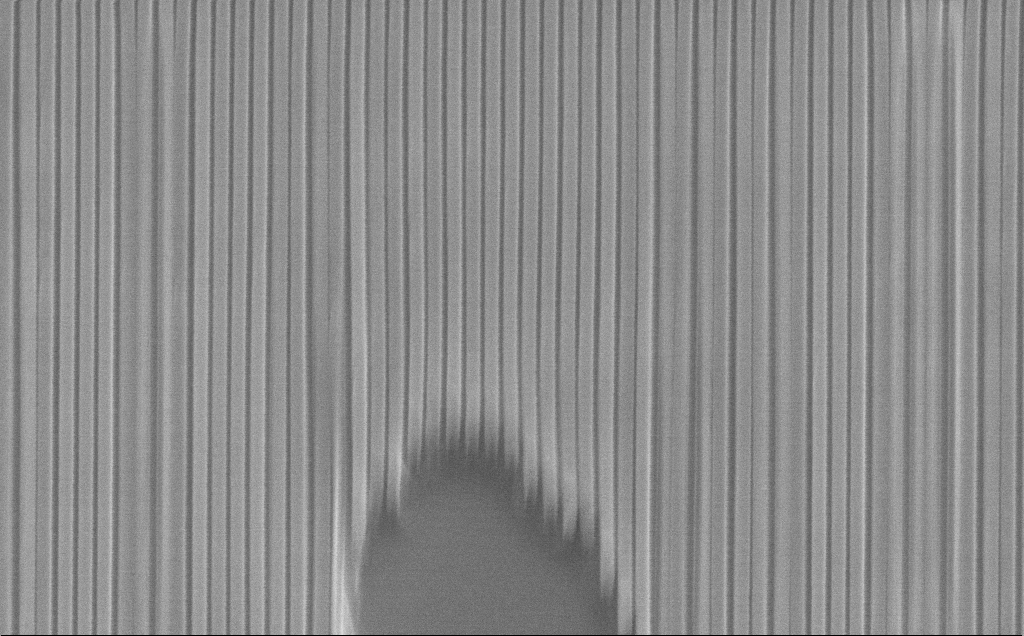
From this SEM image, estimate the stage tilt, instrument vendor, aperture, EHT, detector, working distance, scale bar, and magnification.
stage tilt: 45°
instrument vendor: Zeiss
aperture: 30 µm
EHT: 10 kV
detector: SE2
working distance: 10 mm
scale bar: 1000 nm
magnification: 39.68 K X